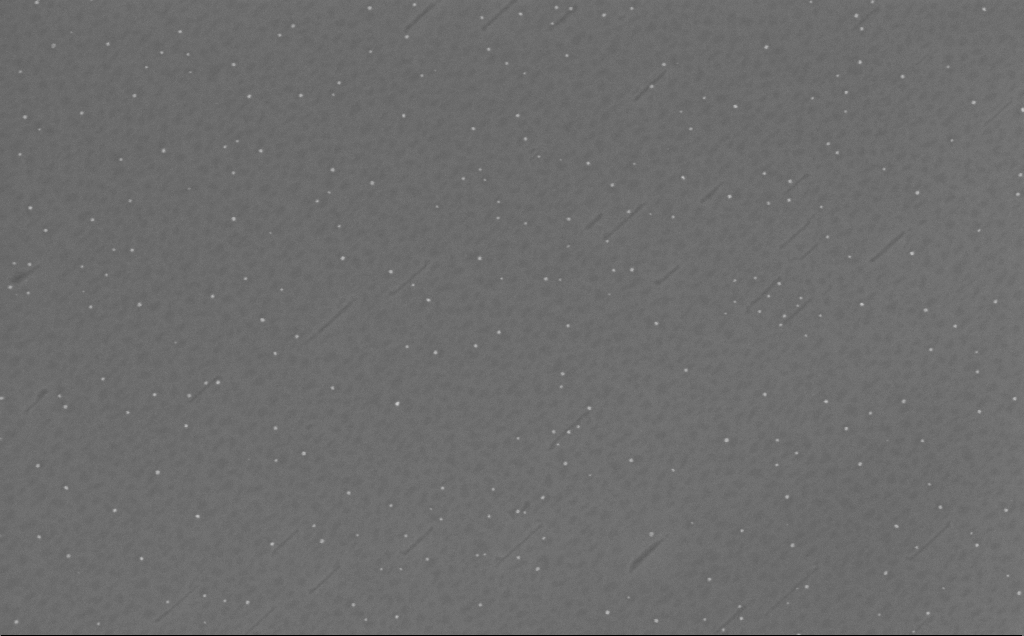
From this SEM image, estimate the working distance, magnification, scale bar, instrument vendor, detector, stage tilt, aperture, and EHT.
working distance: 6 mm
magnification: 162.49 K X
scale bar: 200 nm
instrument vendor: Zeiss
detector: InLens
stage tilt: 0°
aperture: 30 µm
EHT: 10 kV